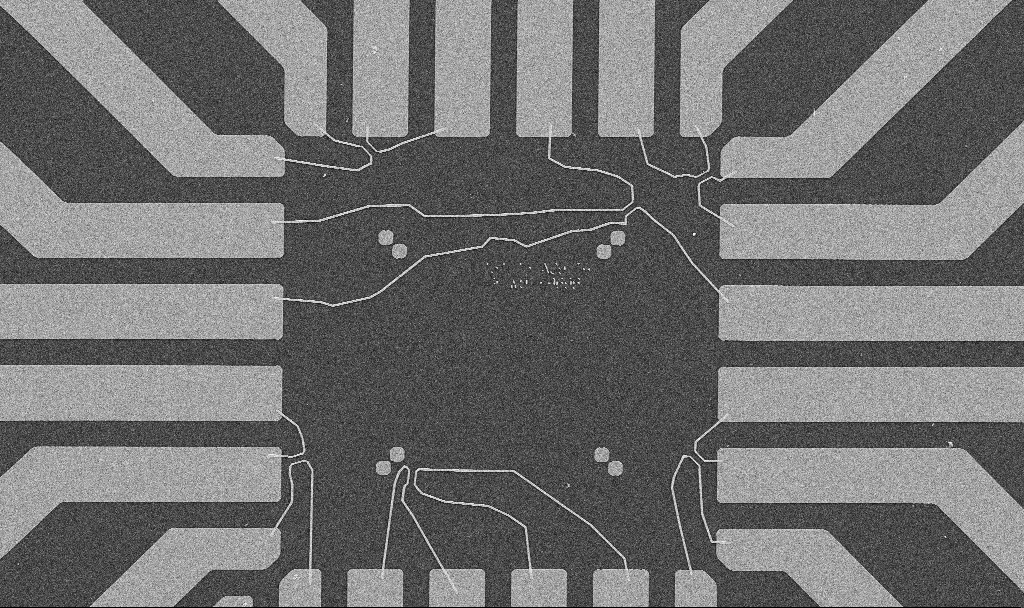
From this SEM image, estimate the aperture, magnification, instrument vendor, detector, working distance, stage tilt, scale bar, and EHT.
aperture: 30 µm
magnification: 1 K X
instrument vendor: Zeiss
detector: SE2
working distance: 10.7 mm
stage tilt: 0°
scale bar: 20000 nm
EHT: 10 kV